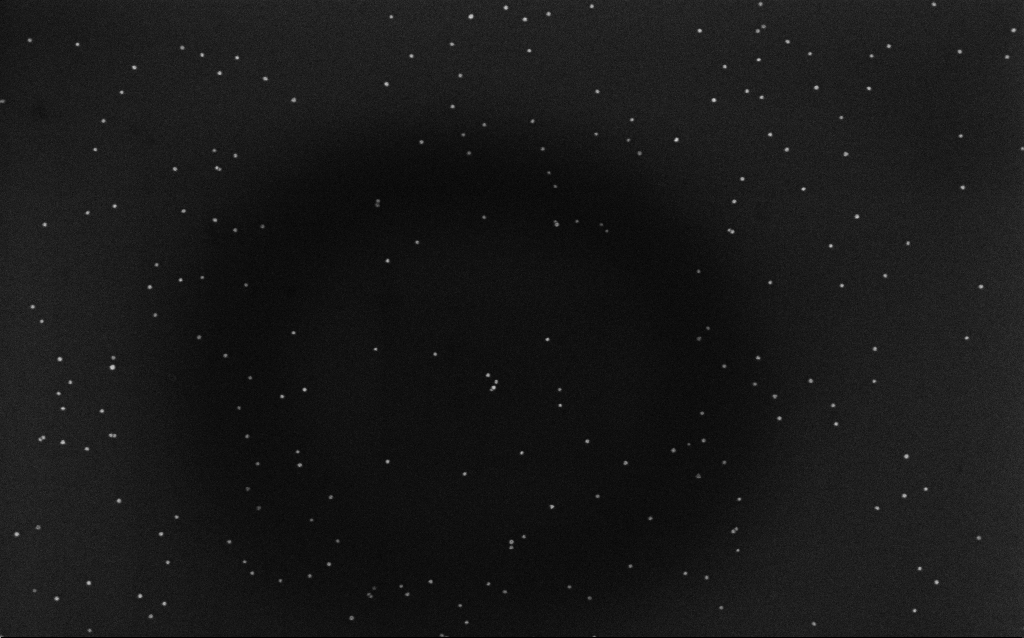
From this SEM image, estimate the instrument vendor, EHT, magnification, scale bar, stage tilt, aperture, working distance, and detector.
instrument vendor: Zeiss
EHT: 10 kV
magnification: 100 K X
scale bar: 200 nm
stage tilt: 0°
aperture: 30 µm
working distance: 6.5 mm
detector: InLens